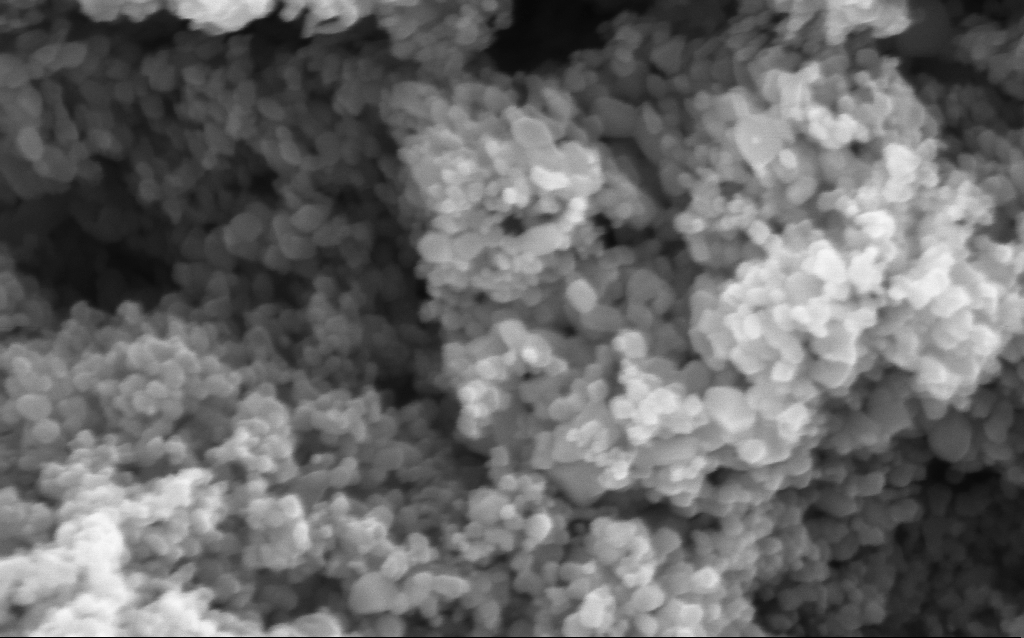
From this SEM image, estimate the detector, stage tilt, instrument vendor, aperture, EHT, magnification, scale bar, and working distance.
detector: InLens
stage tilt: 0°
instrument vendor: Zeiss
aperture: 30 µm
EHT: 5 kV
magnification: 295.08 K X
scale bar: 200 nm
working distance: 4.6 mm